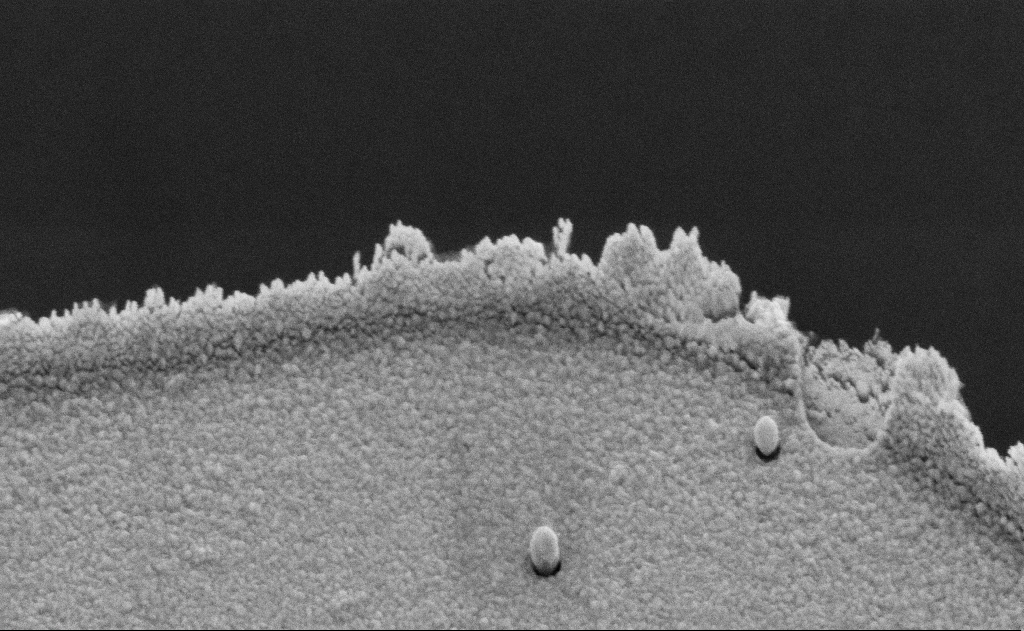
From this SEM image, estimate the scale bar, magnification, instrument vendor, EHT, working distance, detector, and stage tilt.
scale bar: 1000 nm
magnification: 67.85 K X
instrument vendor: Zeiss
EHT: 5 kV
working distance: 10 mm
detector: SE2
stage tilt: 45°